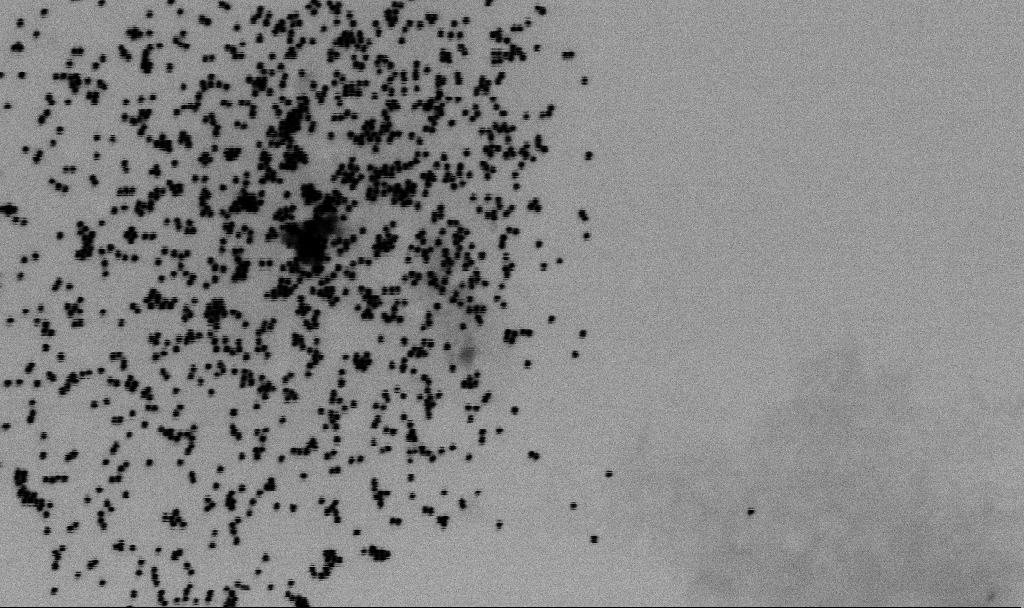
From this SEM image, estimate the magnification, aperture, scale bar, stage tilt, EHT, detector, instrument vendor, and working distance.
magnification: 105.92 K X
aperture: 30 µm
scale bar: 200 nm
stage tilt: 0°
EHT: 2 kV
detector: SE2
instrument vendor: Zeiss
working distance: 6.5 mm